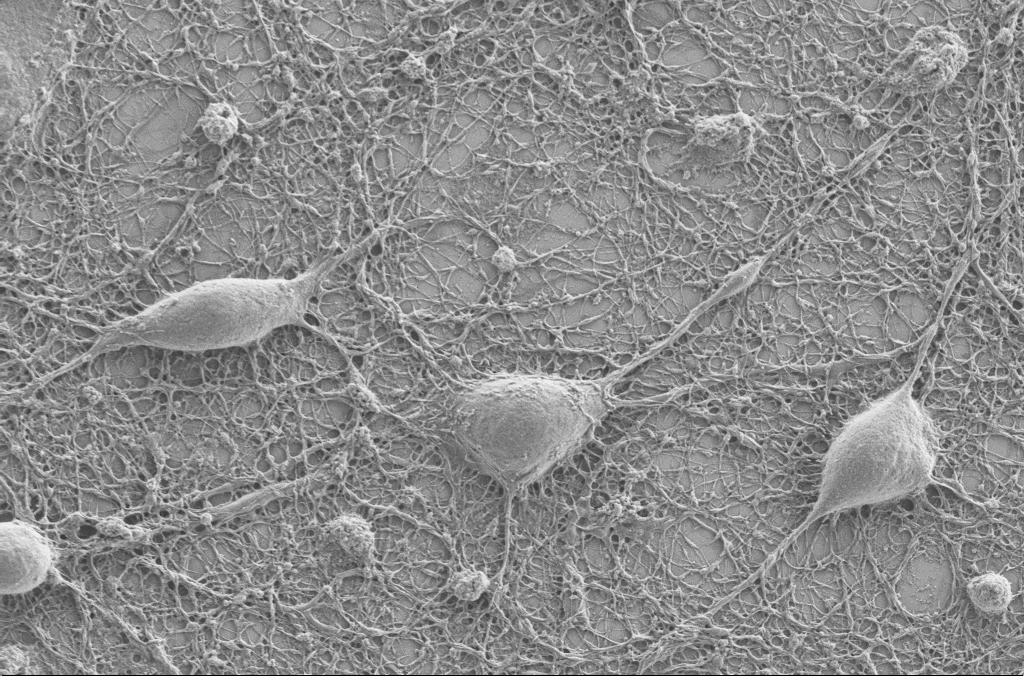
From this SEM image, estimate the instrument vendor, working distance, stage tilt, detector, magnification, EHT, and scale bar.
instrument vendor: Zeiss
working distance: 4 mm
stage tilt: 0°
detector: SE2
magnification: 5 K X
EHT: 2 kV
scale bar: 10000 nm